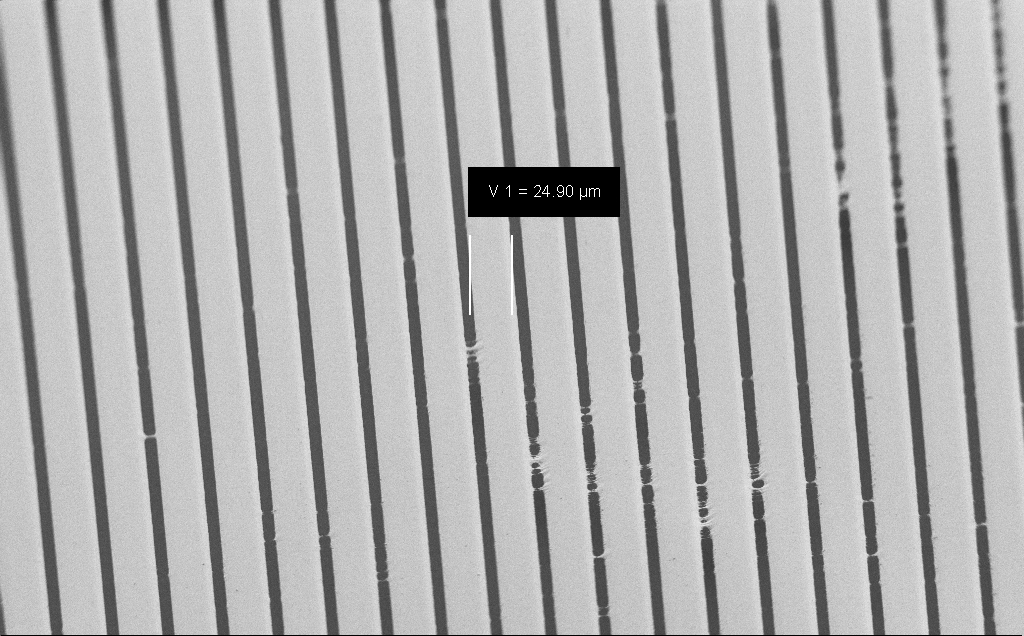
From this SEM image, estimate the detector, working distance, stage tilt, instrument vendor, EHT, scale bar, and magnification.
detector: SE2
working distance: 8 mm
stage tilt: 45°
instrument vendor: Zeiss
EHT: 1.2 kV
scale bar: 100000 nm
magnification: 0.619 K X